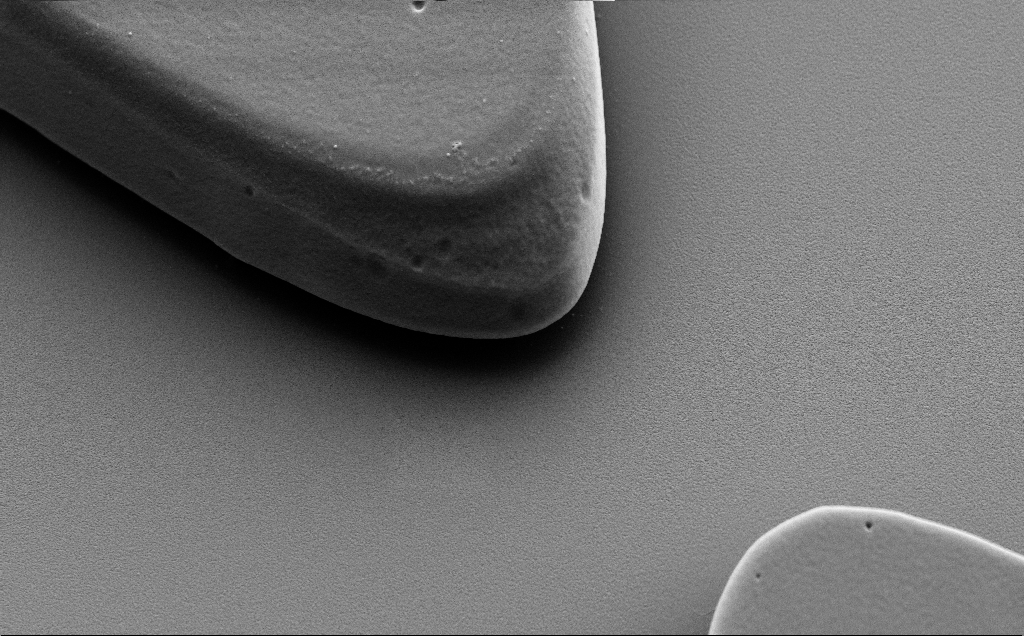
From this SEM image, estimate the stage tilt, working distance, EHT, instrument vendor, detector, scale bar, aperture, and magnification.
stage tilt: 40°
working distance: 9 mm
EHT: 5 kV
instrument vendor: Zeiss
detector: SE2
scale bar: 2000 nm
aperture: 30 µm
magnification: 10 K X